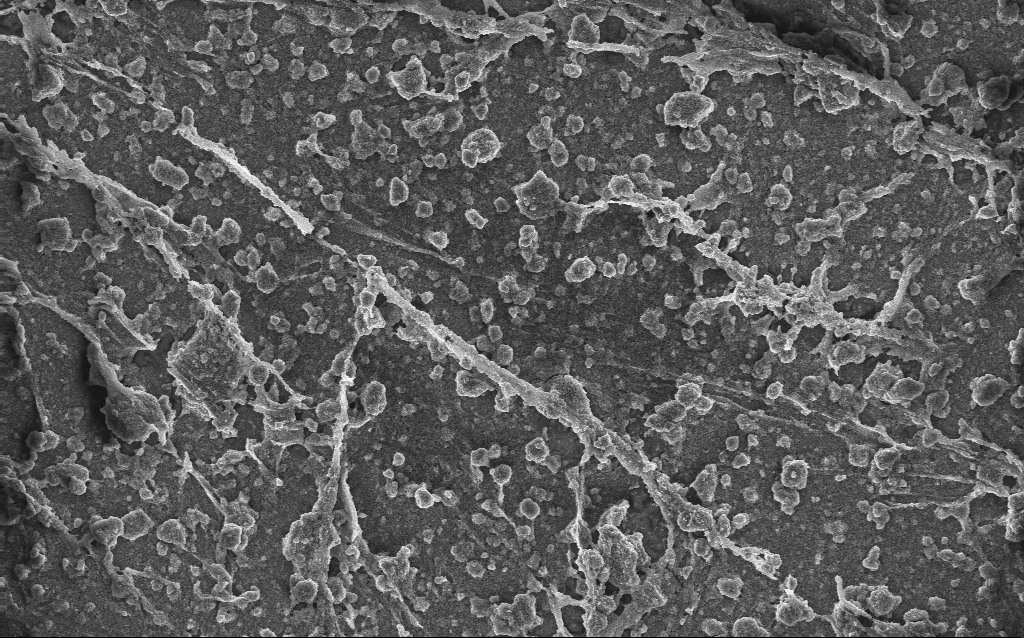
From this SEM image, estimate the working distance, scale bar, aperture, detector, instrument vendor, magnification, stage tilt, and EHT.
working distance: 2.7 mm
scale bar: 10000 nm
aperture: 30 µm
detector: InLens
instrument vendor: Zeiss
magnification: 1.69 K X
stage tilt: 0°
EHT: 10 kV